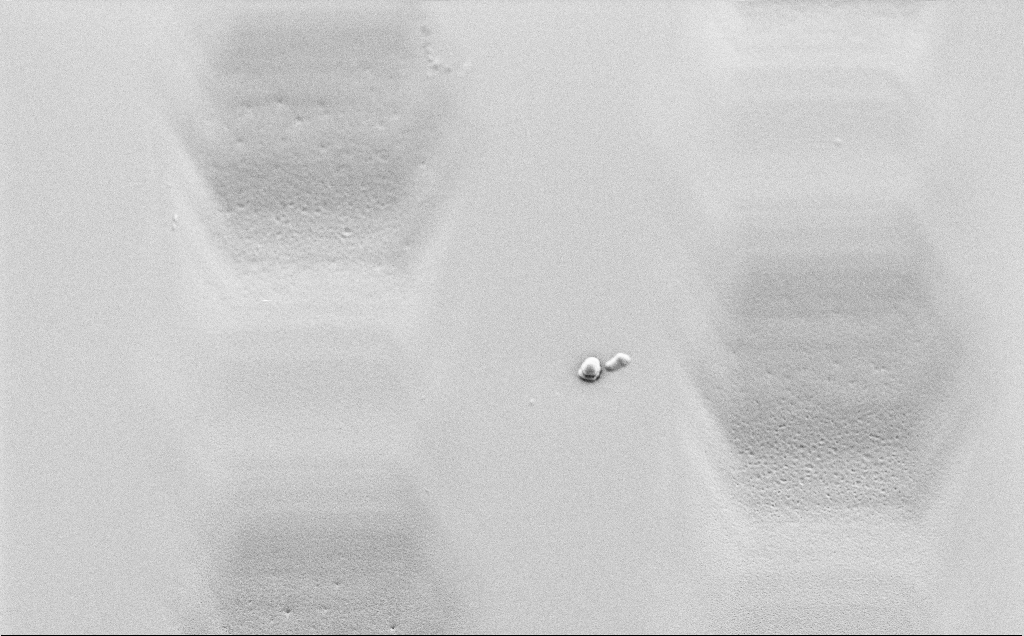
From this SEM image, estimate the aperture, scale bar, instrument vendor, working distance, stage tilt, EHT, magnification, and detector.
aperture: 30 µm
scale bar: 10000 nm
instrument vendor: Zeiss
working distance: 6 mm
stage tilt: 30°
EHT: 1.5 kV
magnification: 5.42 K X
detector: SE2